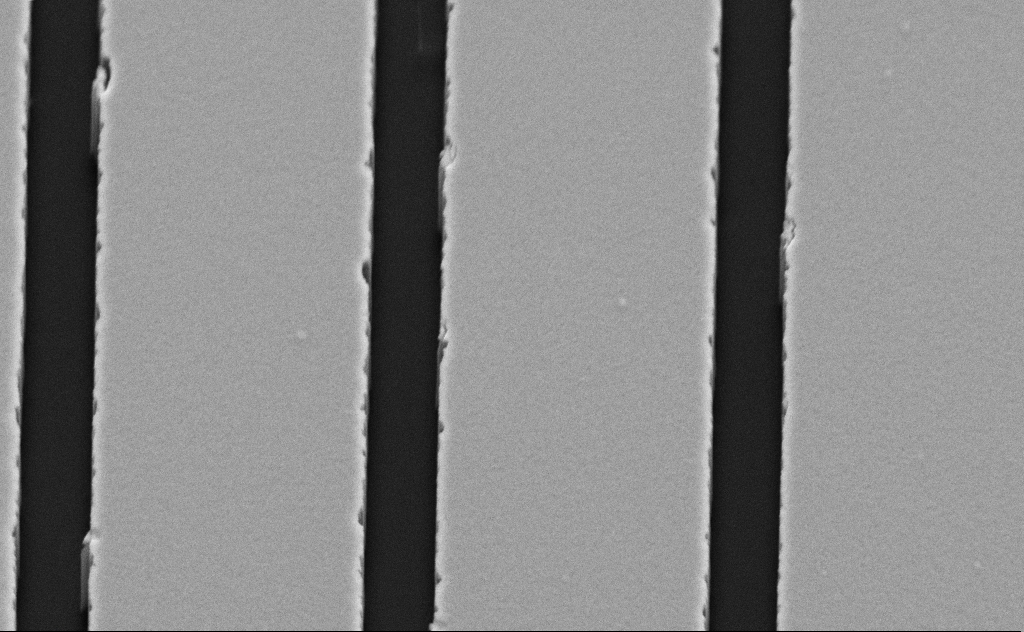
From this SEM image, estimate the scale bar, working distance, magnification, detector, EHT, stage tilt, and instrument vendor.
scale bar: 2000 nm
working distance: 9 mm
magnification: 31.56 K X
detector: SE2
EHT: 5 kV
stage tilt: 45°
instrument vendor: Zeiss